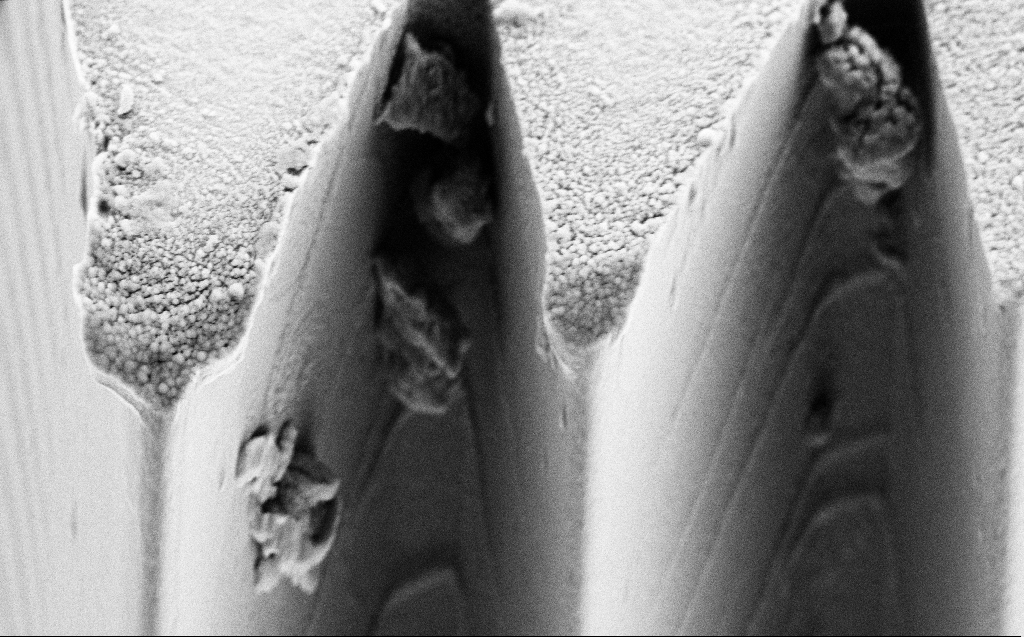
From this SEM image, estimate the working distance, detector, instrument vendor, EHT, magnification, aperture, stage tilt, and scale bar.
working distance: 4 mm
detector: SE2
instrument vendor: Zeiss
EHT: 5 kV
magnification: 14.28 K X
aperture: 30 µm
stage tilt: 45°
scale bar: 2000 nm